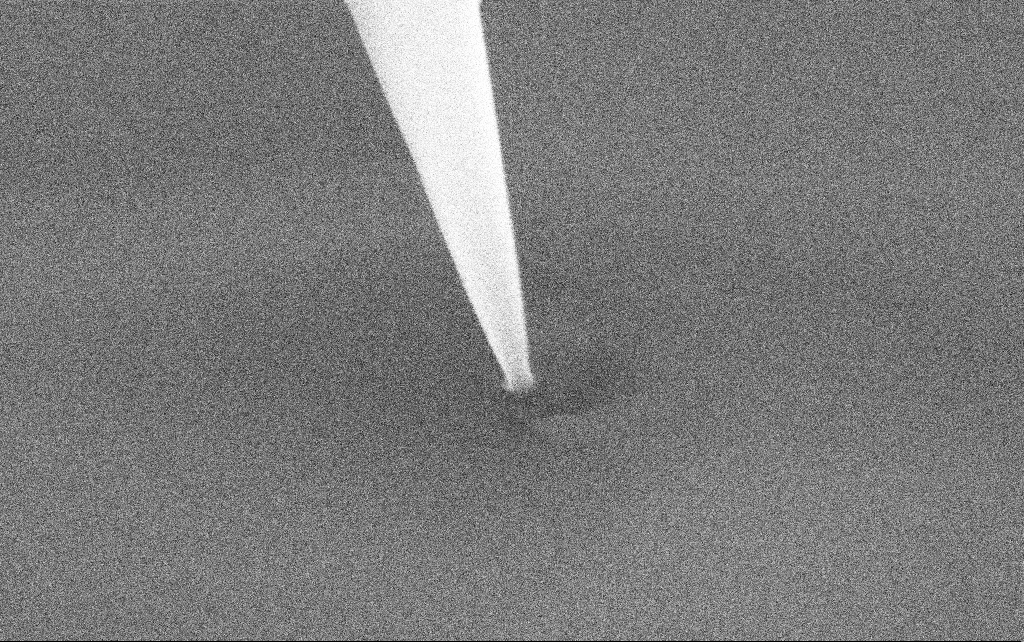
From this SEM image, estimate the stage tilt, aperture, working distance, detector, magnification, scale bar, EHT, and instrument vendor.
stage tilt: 0°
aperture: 30 µm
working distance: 6.7 mm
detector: SE2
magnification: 150 K X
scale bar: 200 nm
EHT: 3 kV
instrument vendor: Zeiss